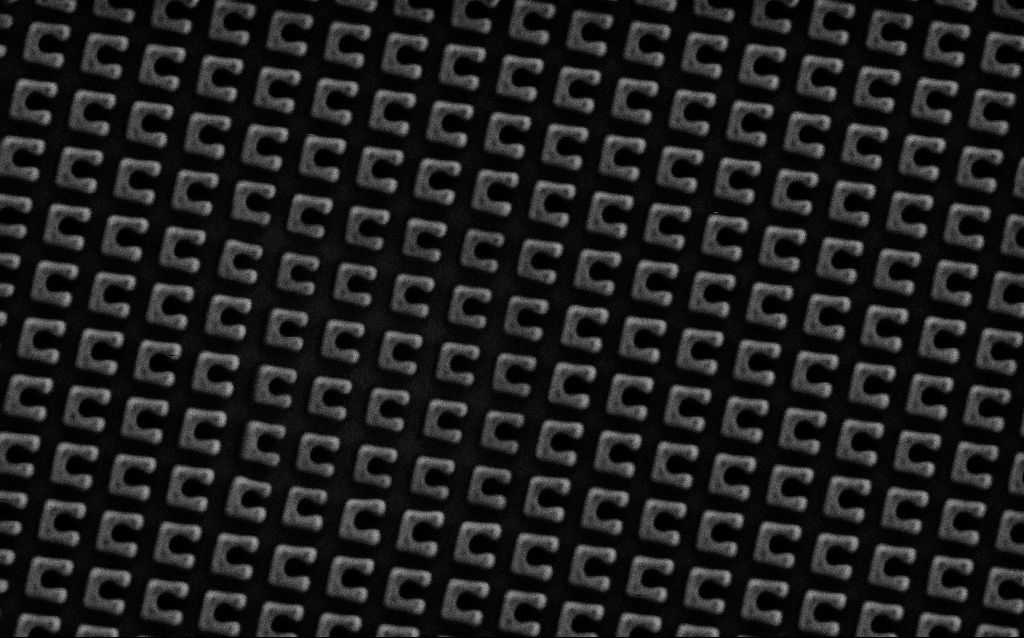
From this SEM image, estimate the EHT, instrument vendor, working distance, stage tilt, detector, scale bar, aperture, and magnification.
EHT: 1.5 kV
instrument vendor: Zeiss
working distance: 7.7 mm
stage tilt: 0°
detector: SE2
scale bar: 1000 nm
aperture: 30 µm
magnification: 46.34 K X